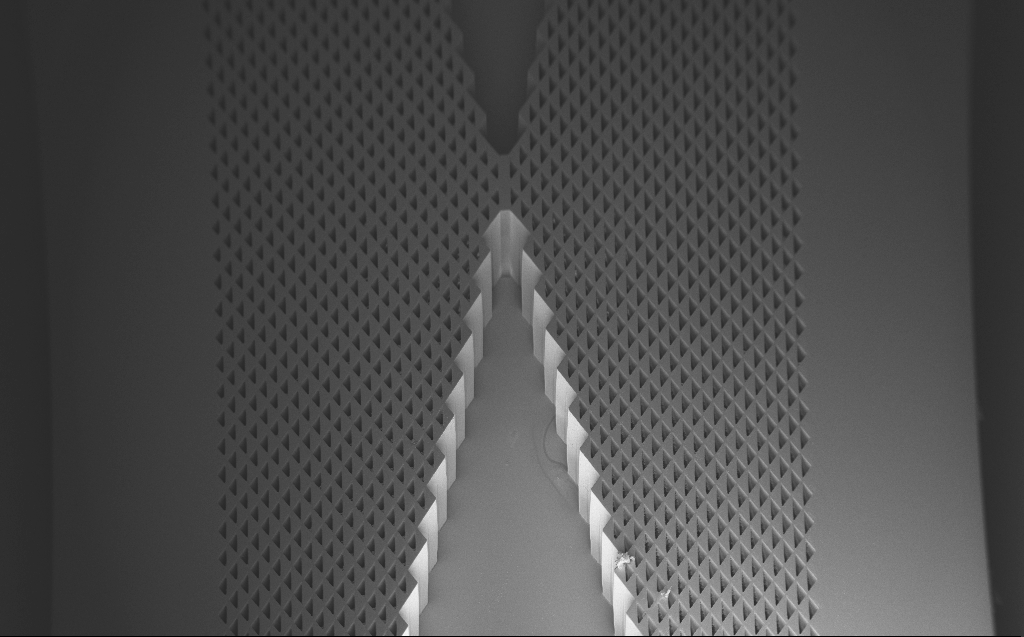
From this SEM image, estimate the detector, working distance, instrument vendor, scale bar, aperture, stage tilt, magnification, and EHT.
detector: InLens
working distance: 7 mm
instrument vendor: Zeiss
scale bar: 200000 nm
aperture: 30 µm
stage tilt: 45°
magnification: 0.108 K X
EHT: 3 kV